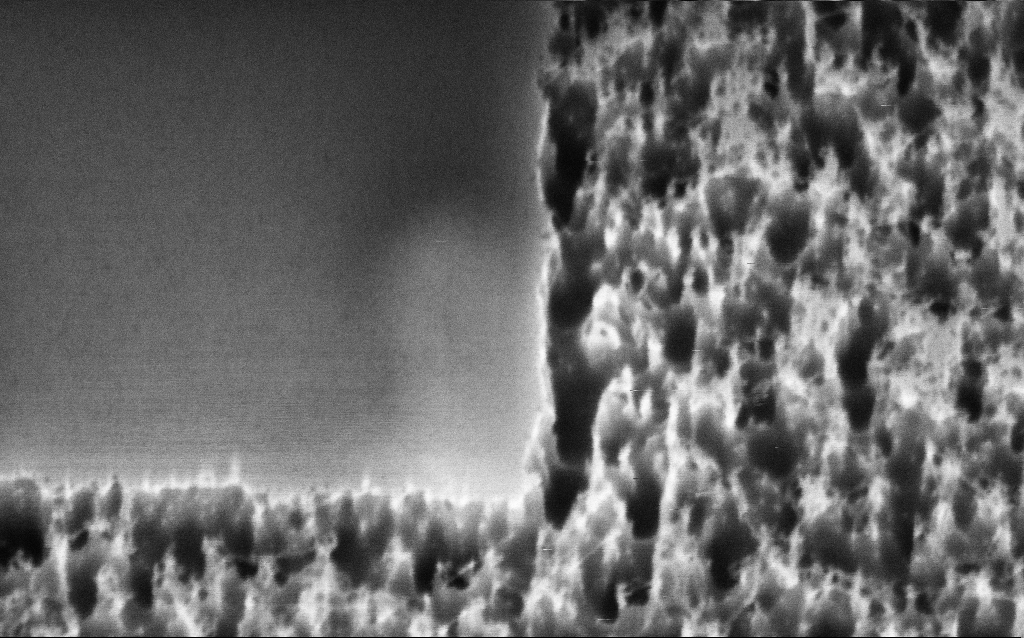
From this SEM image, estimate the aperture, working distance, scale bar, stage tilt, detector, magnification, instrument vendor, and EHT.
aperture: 30 µm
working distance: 7 mm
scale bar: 200 nm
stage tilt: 45°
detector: InLens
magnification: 215.36 K X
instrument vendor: Zeiss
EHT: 3 kV